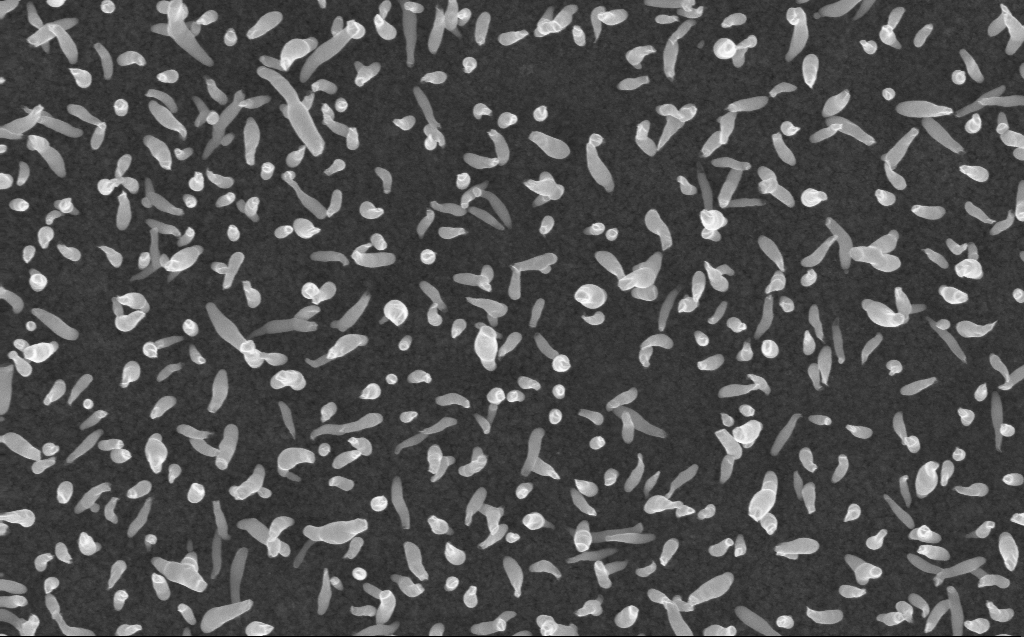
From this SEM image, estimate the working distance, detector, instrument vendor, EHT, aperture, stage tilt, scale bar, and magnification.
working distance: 3 mm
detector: InLens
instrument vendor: Zeiss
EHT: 10 kV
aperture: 30 µm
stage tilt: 0°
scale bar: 1000 nm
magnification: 50 K X